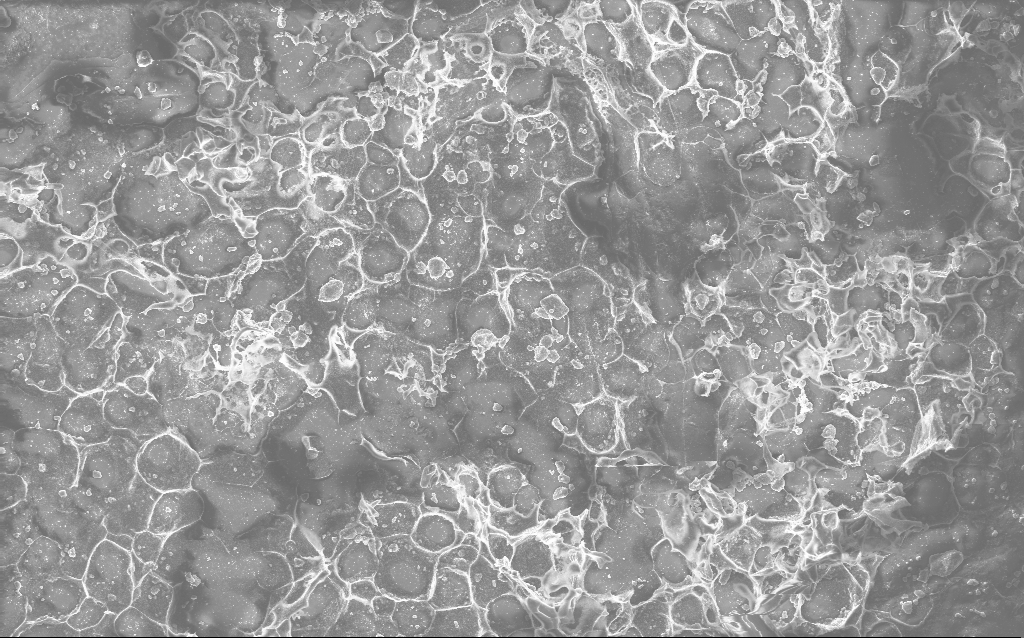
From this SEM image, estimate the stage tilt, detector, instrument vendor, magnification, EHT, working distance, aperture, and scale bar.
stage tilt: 0°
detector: InLens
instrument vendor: Zeiss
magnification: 0.792 K X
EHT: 10 kV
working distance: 2.8 mm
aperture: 30 µm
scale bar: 20000 nm